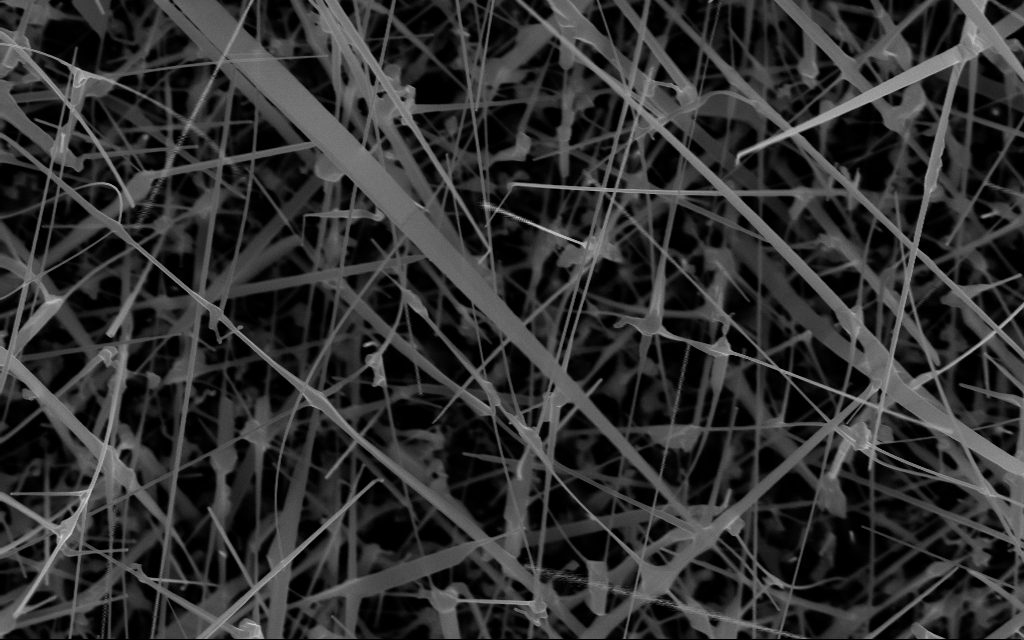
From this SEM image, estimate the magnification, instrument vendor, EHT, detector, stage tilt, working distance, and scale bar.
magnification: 40 K X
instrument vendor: Zeiss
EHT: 10 kV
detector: InLens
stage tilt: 0°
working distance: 7 mm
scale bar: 1000 nm